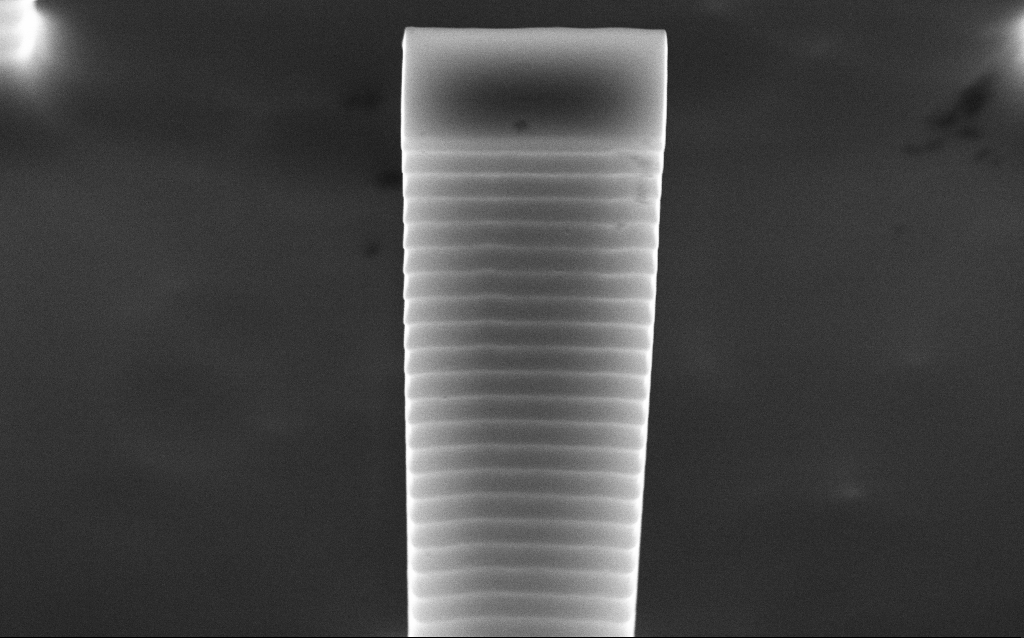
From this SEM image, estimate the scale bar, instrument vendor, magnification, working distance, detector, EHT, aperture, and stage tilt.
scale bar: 1000 nm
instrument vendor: Zeiss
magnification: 37.49 K X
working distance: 5.1 mm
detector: InLens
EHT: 10 kV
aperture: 30 µm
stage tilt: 45°